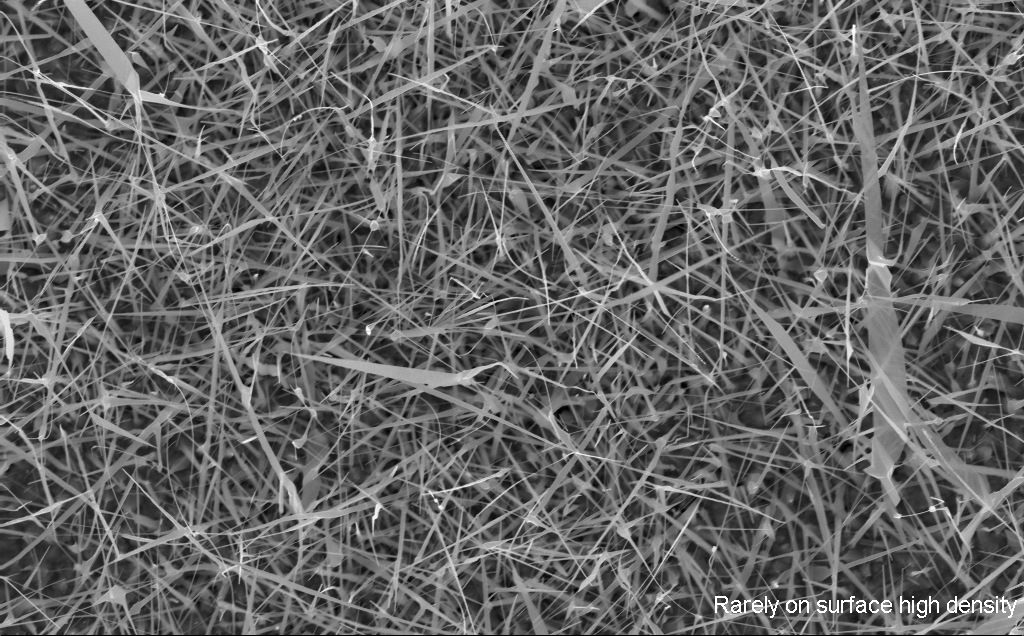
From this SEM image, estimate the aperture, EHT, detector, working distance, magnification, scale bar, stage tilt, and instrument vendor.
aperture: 30 µm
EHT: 10 kV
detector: InLens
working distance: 6 mm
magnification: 20 K X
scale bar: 2000 nm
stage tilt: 0°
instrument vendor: Zeiss